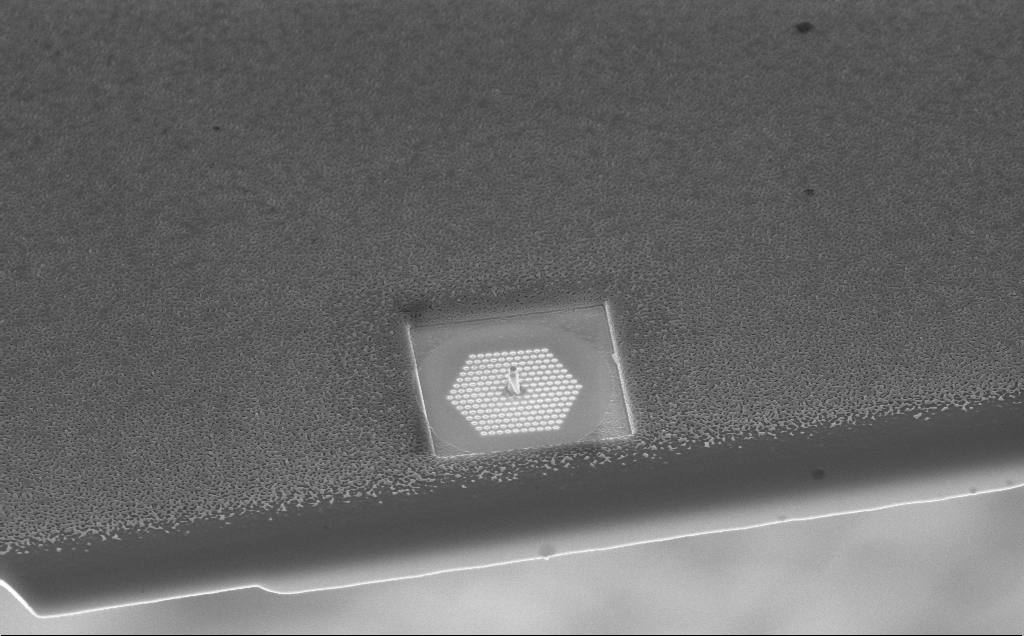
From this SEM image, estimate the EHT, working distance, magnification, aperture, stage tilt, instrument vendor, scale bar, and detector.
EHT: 10 kV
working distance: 8 mm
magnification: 11.99 K X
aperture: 30 µm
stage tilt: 45°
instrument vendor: Zeiss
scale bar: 1000 nm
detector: InLens